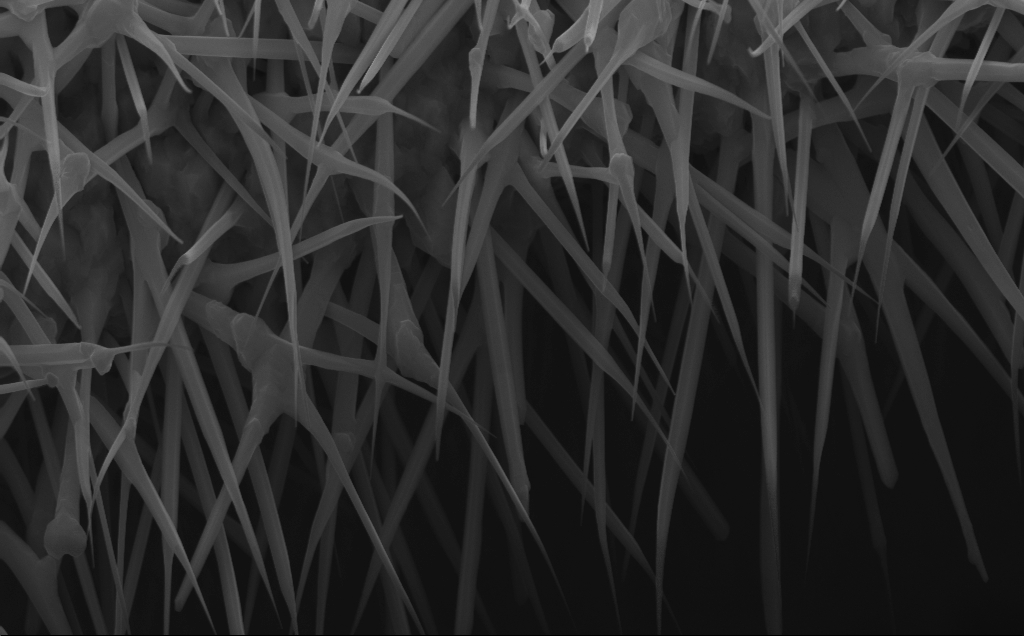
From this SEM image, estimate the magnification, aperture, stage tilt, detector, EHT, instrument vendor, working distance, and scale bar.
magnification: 40 K X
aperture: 30 µm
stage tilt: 0°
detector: InLens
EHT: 10 kV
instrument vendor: Zeiss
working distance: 7 mm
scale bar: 1000 nm